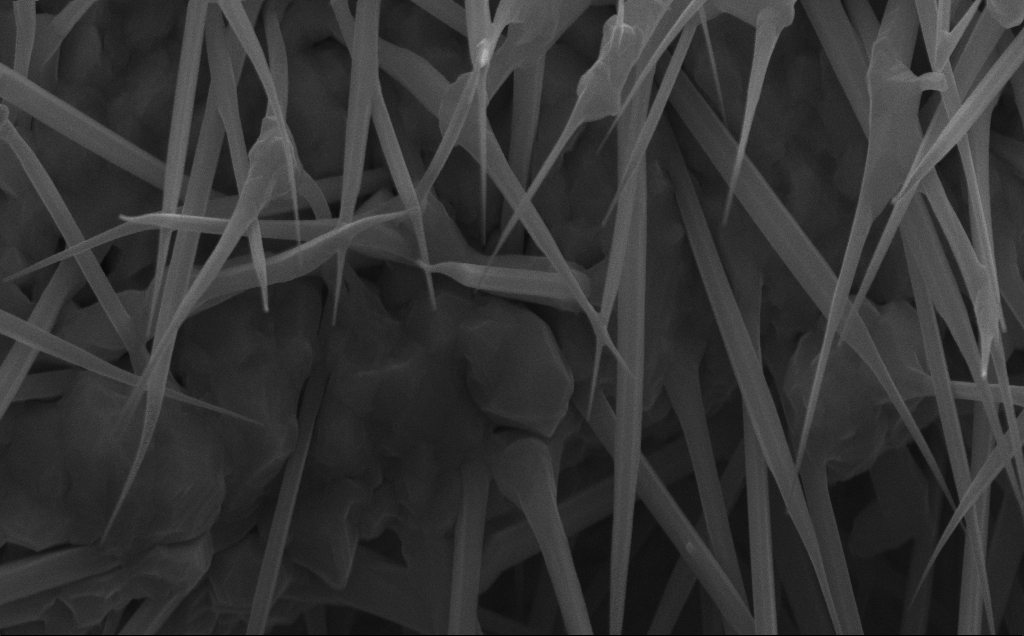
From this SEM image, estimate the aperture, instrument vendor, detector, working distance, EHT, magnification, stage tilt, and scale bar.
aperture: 30 µm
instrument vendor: Zeiss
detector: InLens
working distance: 7 mm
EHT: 10 kV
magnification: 65.73 K X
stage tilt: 0°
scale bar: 1000 nm